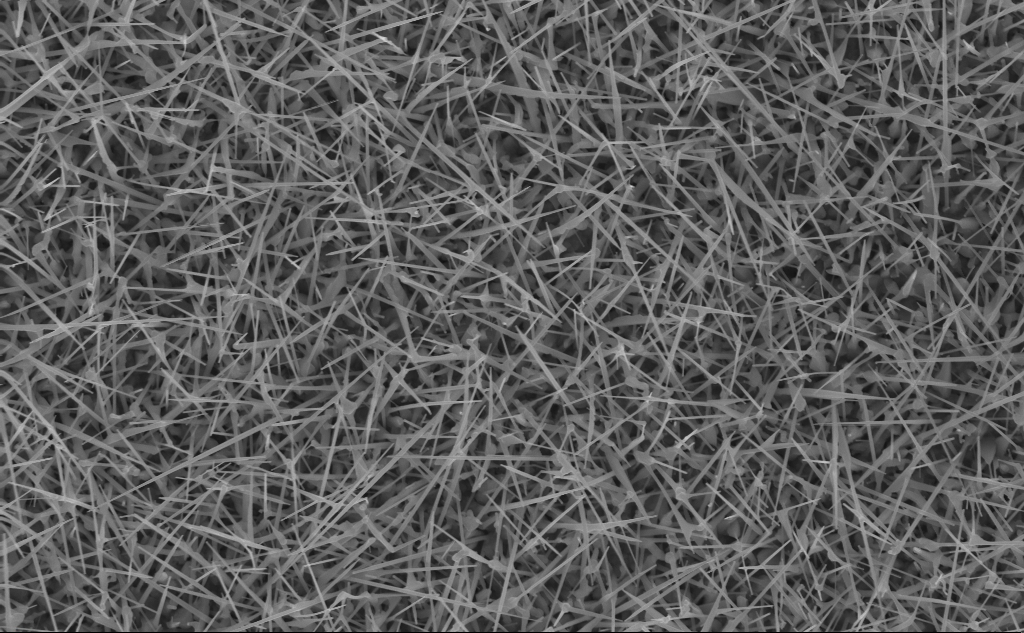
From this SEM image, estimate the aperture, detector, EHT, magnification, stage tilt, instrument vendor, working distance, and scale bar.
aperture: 30 µm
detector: InLens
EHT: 10 kV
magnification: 20 K X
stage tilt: -0.2°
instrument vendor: Zeiss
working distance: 7 mm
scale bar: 2000 nm